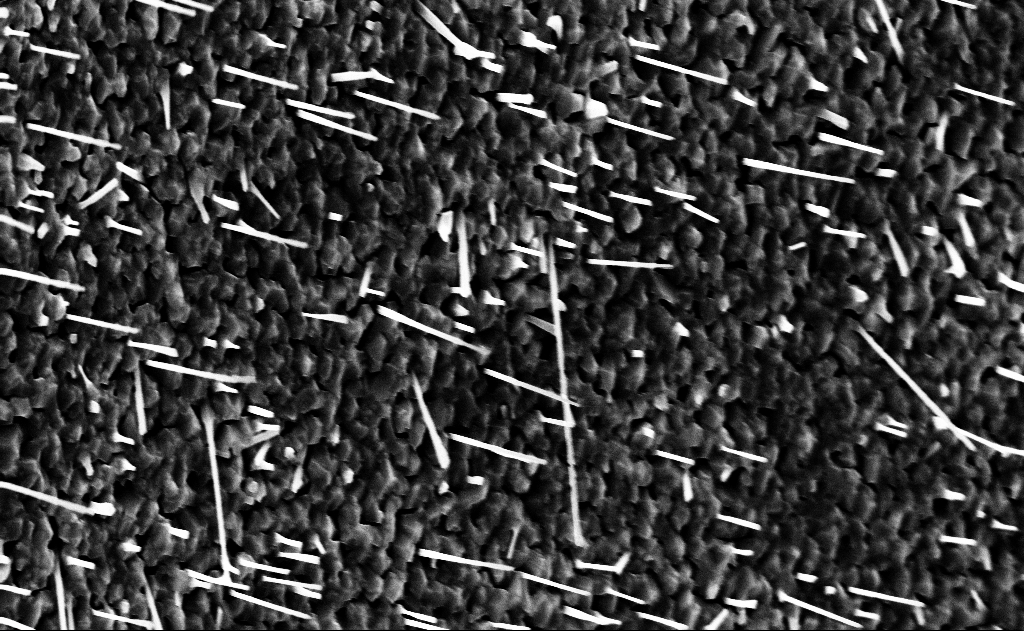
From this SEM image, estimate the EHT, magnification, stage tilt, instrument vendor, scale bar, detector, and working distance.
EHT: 10 kV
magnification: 20 K X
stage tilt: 0°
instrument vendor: Zeiss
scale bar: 2000 nm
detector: InLens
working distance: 14 mm